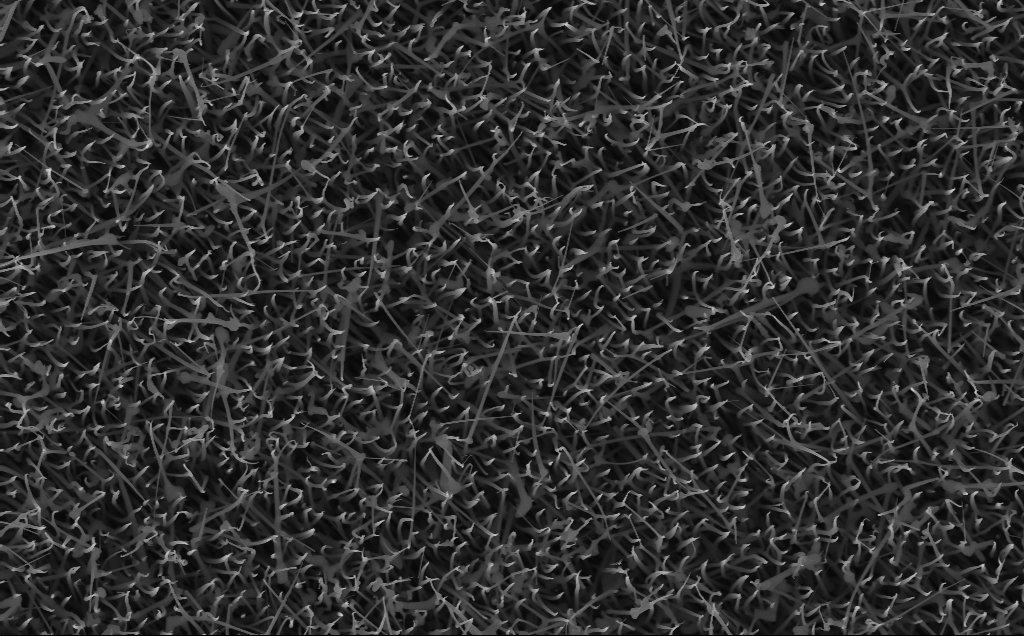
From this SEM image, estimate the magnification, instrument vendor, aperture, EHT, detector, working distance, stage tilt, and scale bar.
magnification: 20 K X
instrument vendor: Zeiss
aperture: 30 µm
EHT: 10 kV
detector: InLens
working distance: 4 mm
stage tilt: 0°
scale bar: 2000 nm